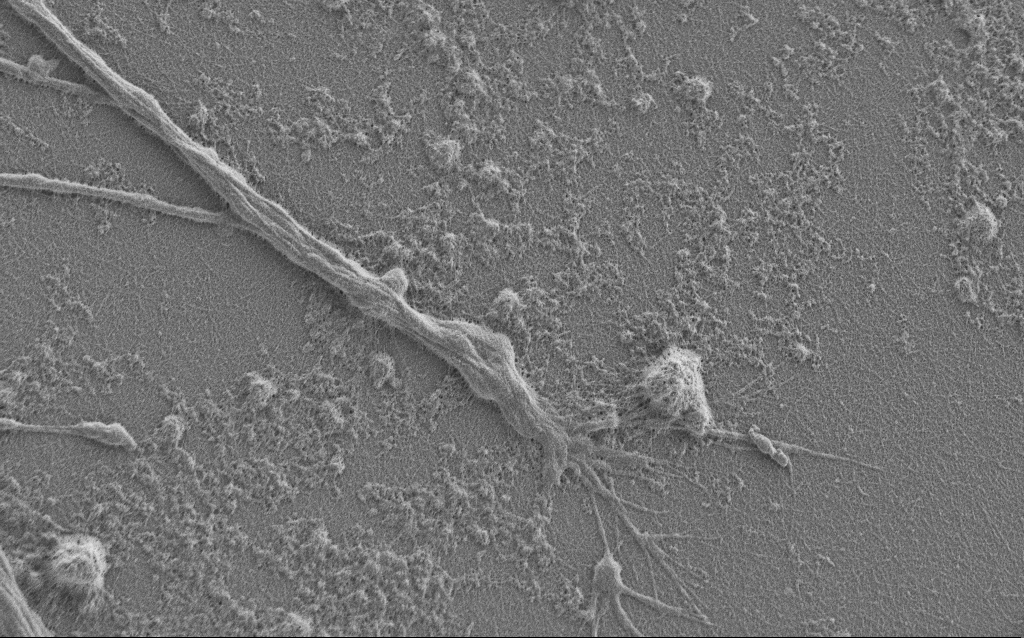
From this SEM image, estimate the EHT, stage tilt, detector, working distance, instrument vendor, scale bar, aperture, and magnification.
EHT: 1 kV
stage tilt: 0°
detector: SE2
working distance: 6 mm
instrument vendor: Zeiss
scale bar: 2000 nm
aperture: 30 µm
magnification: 7.5 K X